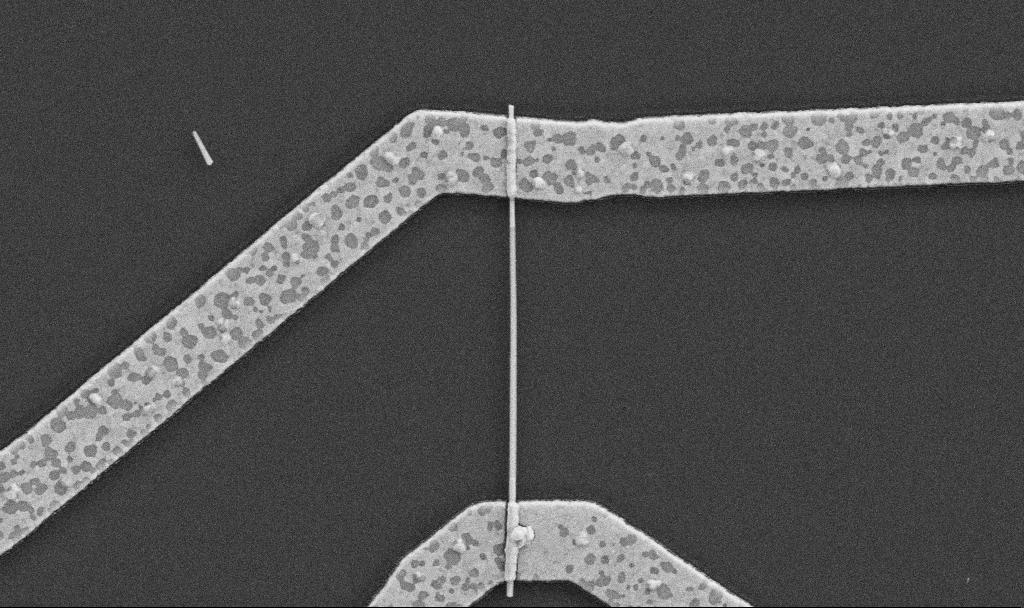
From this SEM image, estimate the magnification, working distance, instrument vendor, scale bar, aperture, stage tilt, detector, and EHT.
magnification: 30 K X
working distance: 8.7 mm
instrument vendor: Zeiss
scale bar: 1000 nm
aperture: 30 µm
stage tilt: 0°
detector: SE2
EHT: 5 kV